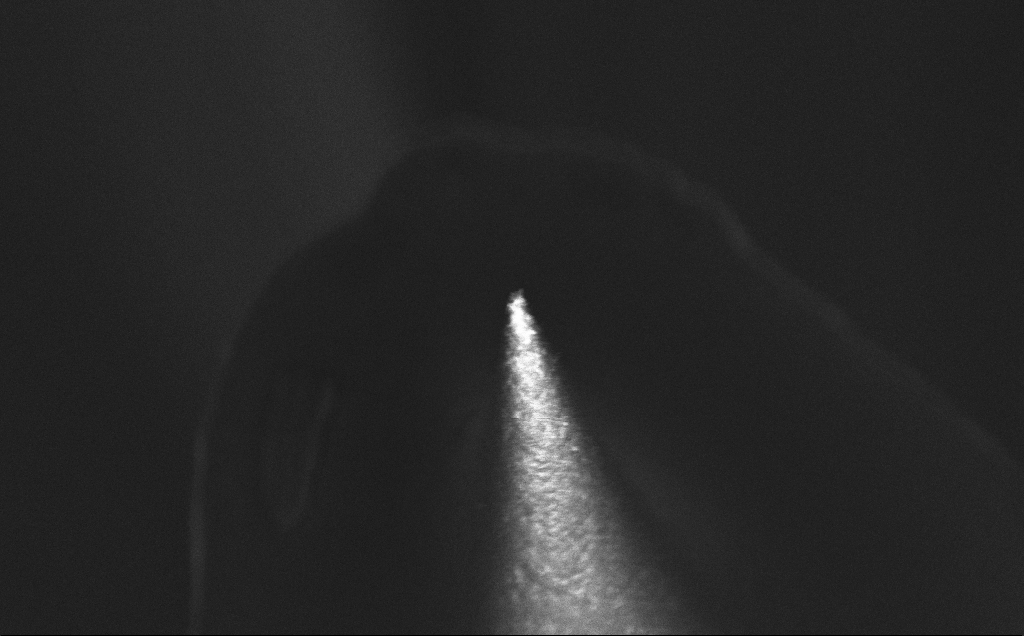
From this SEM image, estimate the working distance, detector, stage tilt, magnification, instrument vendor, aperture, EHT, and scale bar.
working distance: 6 mm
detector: InLens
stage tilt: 20°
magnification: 1.81 K X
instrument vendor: Zeiss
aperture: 30 µm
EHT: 5 kV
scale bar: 10000 nm